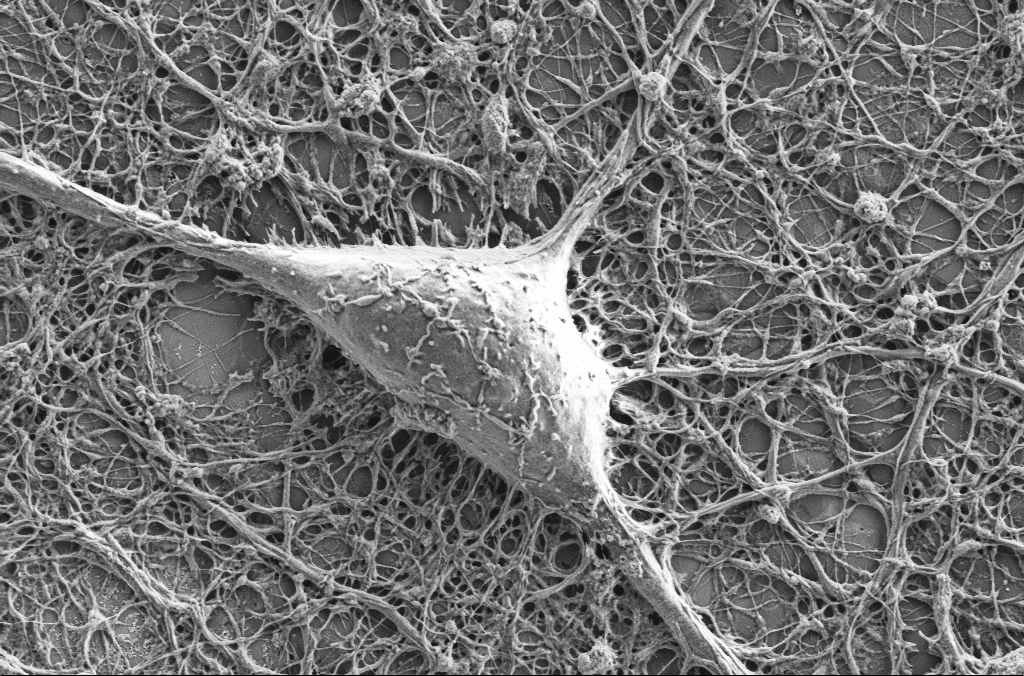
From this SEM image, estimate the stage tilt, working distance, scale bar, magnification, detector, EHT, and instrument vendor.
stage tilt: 0°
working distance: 4.1 mm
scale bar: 2000 nm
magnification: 10 K X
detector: SE2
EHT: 2 kV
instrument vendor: Zeiss